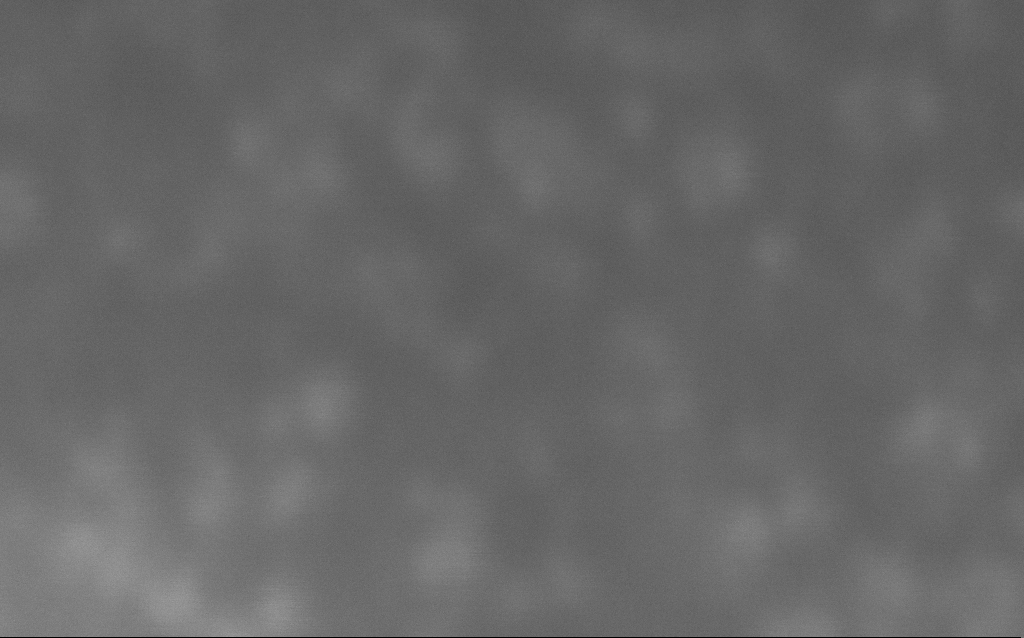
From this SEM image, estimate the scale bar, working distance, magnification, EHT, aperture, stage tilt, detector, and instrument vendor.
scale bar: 20 nm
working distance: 2 mm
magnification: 2989.95 K X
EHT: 10 kV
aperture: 30 µm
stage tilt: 0°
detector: InLens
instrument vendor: Zeiss